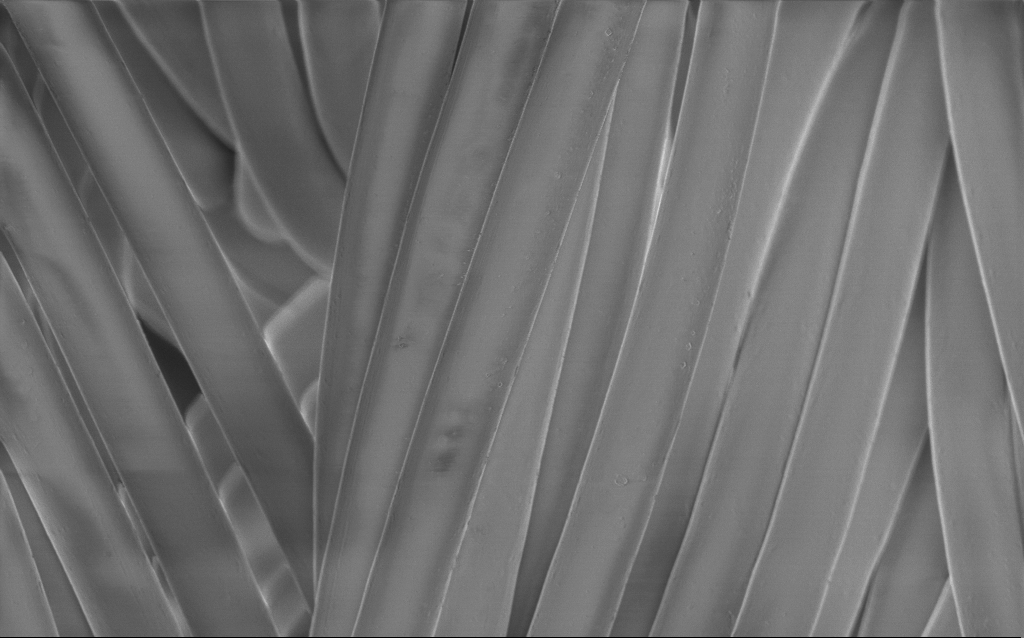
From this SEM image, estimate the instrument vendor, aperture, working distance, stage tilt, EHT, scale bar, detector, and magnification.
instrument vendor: Zeiss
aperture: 30 µm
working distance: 4 mm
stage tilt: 0°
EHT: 1 kV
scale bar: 10000 nm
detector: InLens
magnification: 1.23 K X